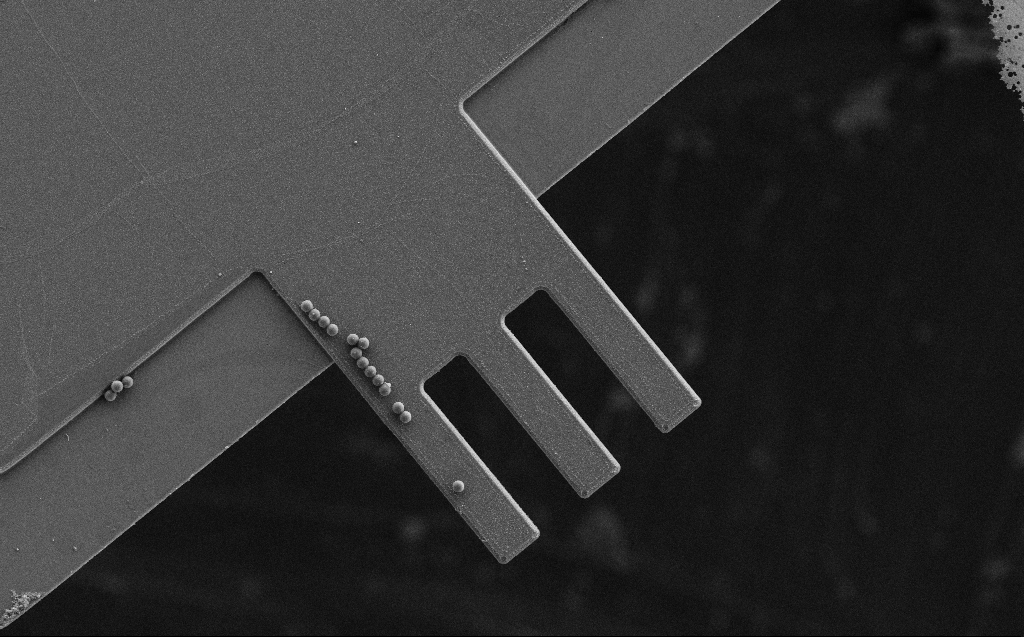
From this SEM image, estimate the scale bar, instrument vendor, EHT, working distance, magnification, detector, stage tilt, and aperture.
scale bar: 20000 nm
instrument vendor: Zeiss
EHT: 10 kV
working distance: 5 mm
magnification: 0.959 K X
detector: SE2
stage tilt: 0°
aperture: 30 µm